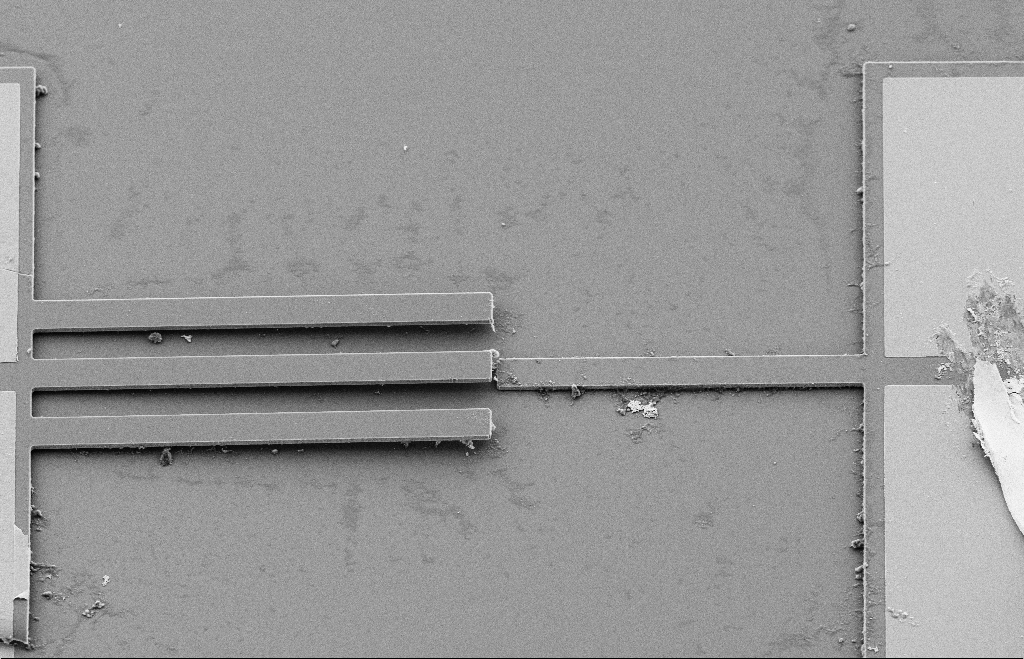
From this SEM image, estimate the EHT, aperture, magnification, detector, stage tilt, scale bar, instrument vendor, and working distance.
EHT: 10 kV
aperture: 20 µm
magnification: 0.675 K X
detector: SE2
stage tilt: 36.3°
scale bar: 20000 nm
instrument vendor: Zeiss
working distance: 10 mm